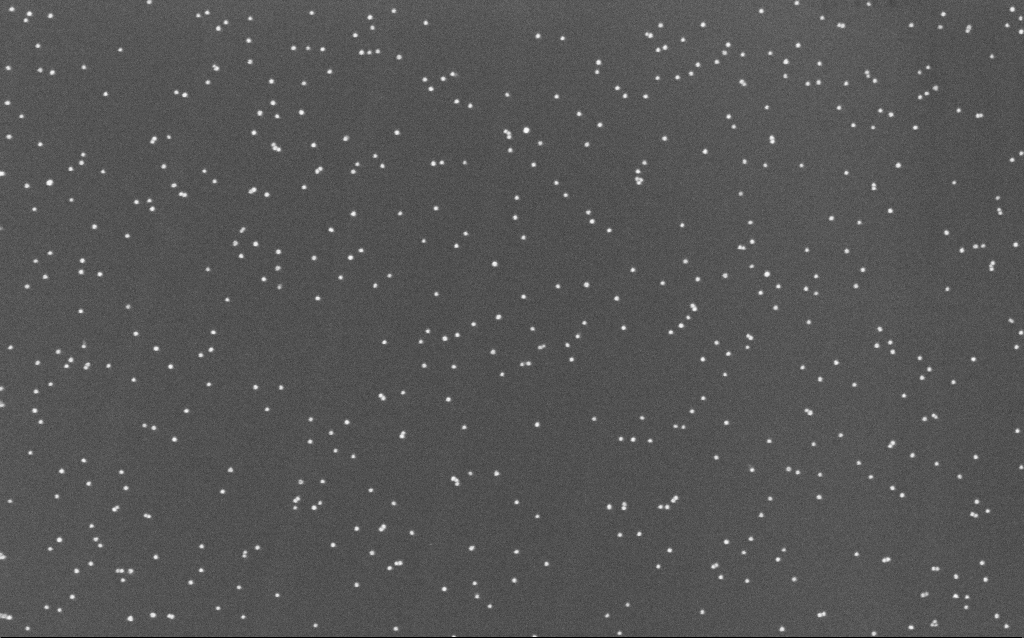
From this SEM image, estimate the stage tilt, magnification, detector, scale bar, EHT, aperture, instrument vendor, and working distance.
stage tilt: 0°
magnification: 100 K X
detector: InLens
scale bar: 200 nm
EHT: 10 kV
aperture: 30 µm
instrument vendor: Zeiss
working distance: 6.6 mm